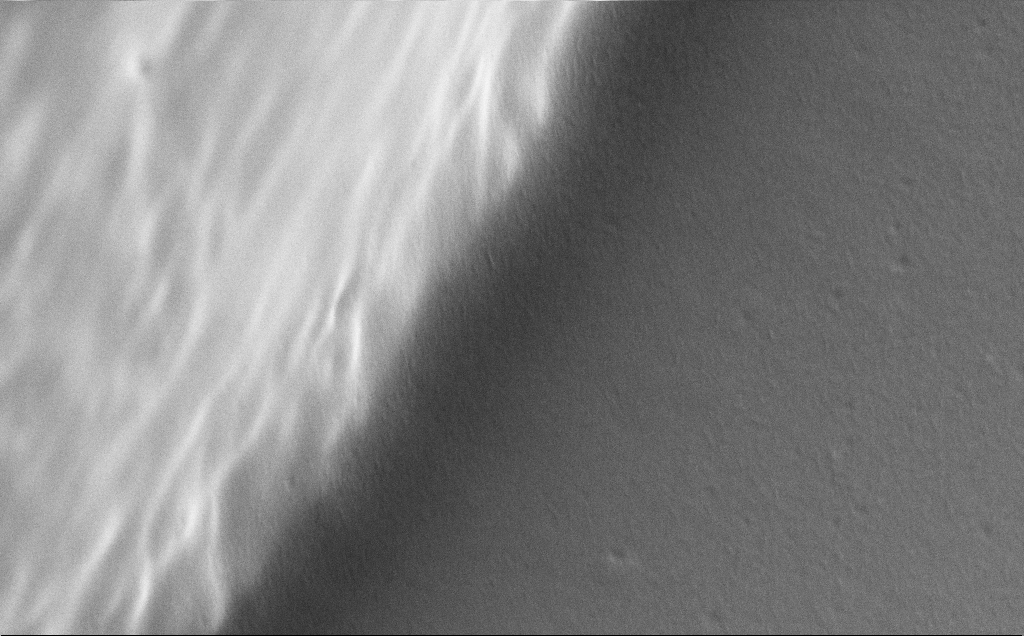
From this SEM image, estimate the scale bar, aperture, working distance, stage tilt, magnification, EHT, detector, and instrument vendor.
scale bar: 200 nm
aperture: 30 µm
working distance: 11 mm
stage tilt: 30°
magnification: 98.02 K X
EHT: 5 kV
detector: SE2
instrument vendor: Zeiss